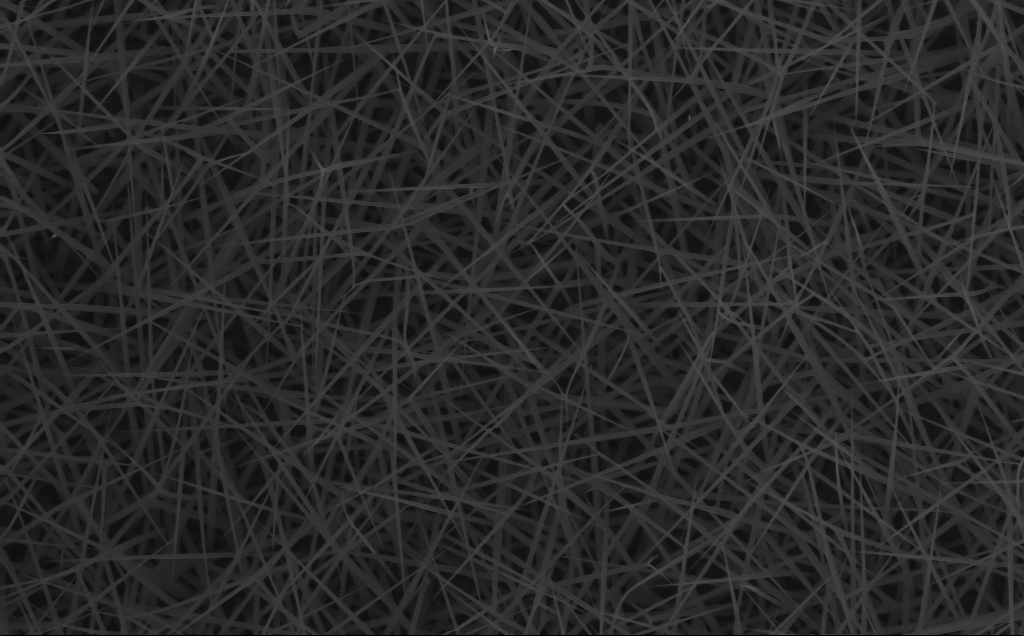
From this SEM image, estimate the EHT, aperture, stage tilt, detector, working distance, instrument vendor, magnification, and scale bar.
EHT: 10 kV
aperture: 30 µm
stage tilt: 0°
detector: InLens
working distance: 4 mm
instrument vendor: Zeiss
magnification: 20 K X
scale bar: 1000 nm